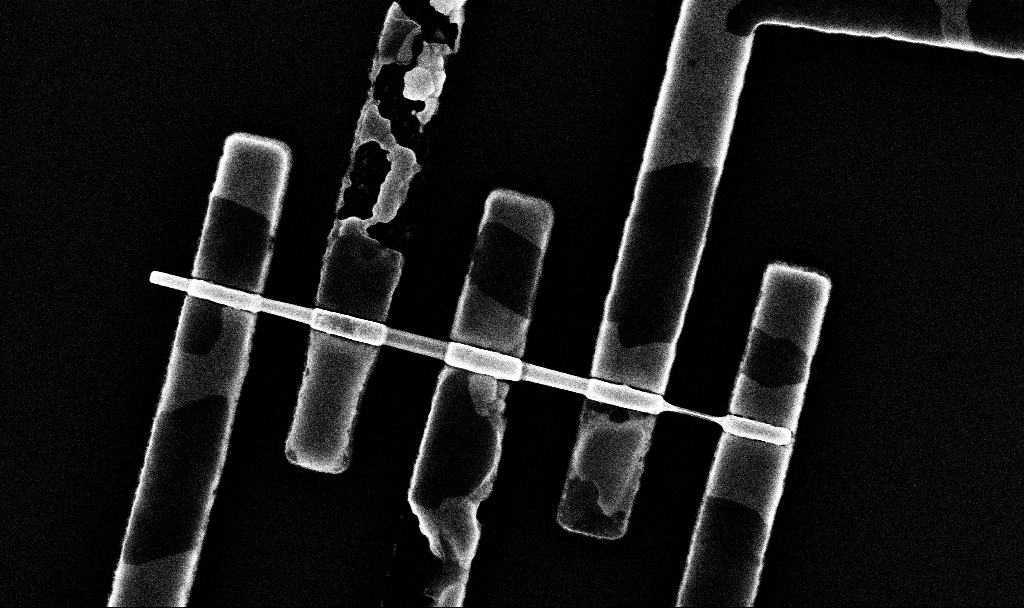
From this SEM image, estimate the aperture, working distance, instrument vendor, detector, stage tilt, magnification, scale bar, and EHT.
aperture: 30 µm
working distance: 6.8 mm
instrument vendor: Zeiss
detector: InLens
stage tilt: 0°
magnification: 44.73 K X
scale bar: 1000 nm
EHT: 10 kV